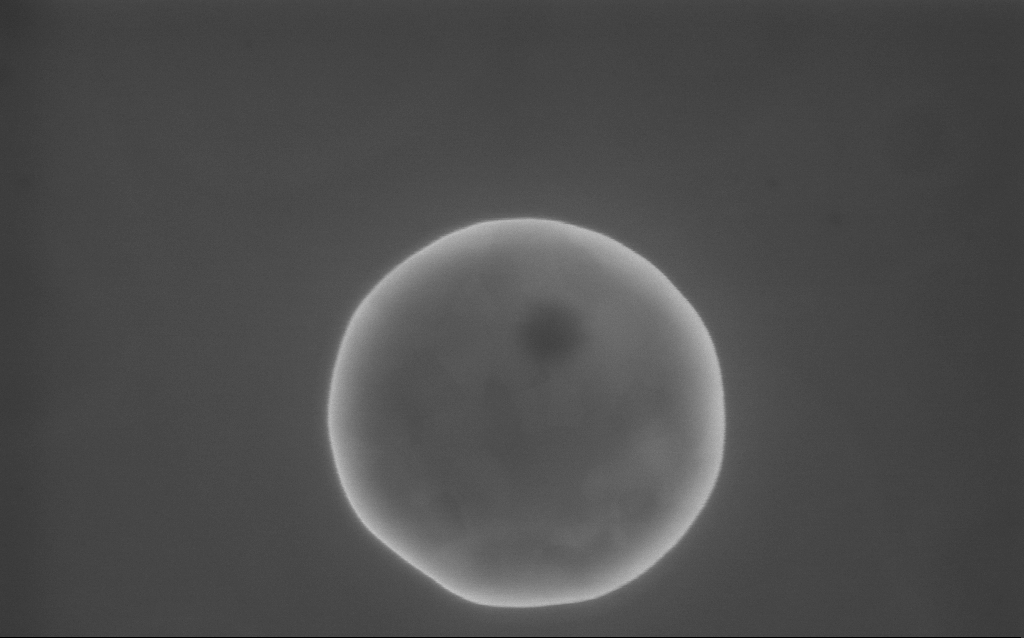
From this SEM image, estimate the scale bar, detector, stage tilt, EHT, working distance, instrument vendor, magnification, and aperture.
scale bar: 200 nm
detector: InLens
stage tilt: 0°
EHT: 10 kV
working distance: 2 mm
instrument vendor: Zeiss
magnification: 114.72 K X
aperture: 30 µm